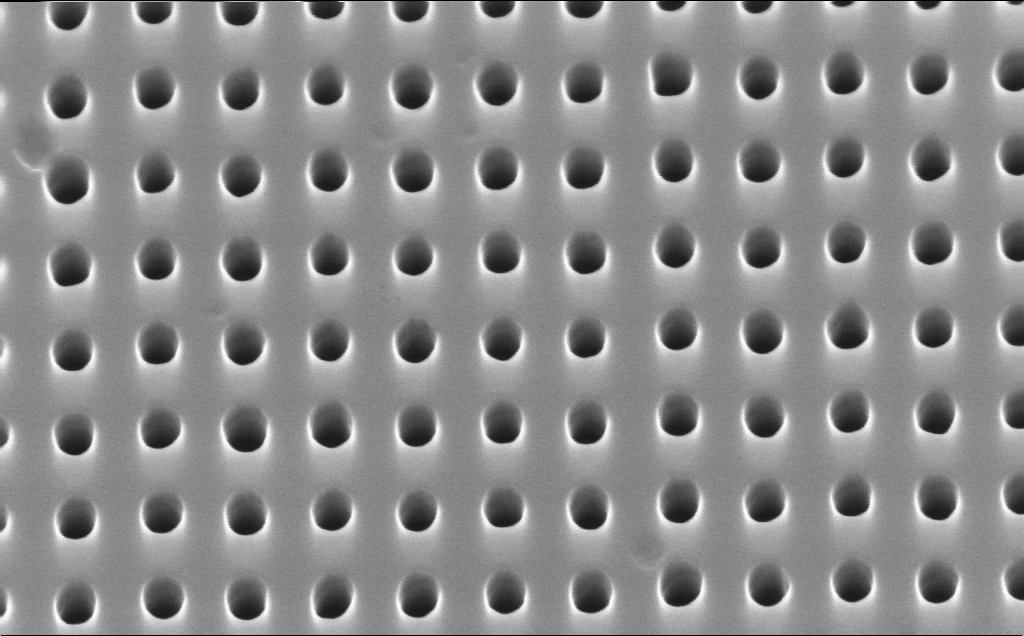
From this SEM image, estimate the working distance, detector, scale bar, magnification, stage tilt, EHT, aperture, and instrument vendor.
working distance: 4 mm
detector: InLens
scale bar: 200 nm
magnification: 158.4 K X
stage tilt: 30°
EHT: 10 kV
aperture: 30 µm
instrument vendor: Zeiss